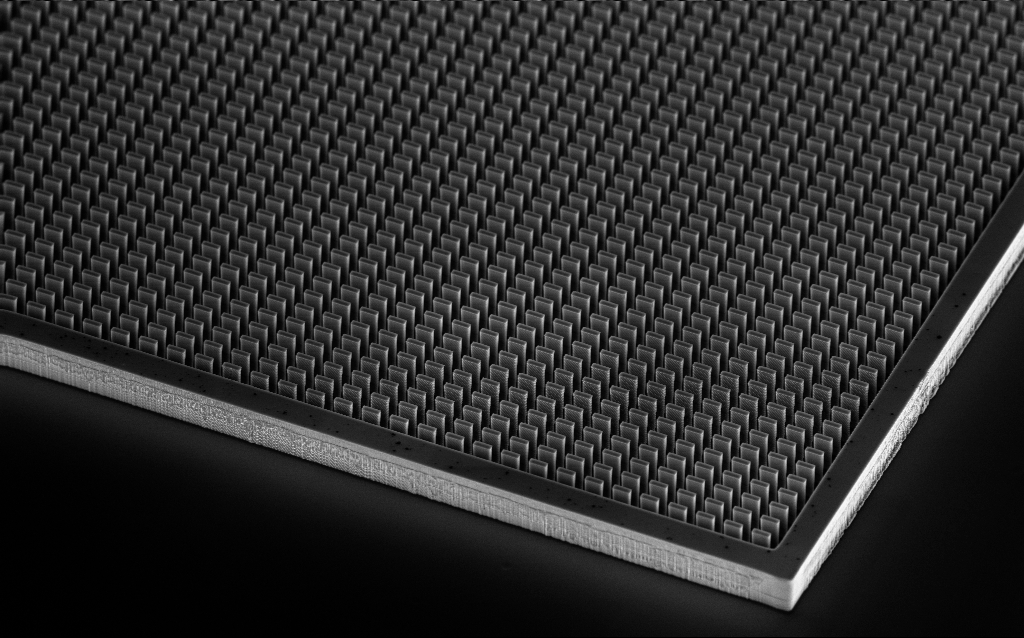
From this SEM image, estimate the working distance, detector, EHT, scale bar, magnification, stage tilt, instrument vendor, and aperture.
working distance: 5.2 mm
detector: InLens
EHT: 10 kV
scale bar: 10000 nm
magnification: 1.42 K X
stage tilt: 45°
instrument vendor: Zeiss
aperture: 30 µm